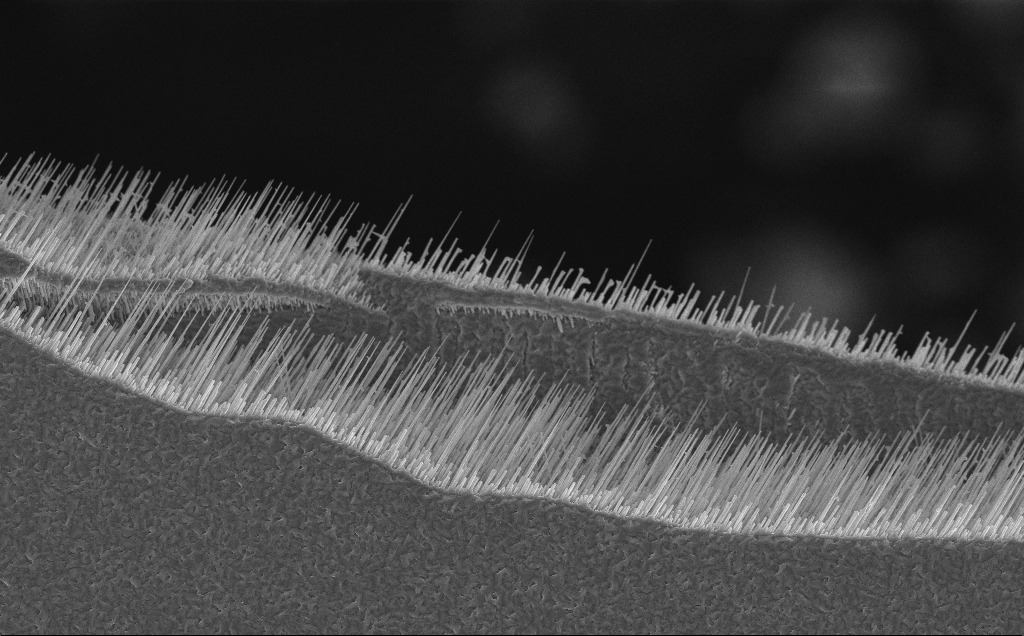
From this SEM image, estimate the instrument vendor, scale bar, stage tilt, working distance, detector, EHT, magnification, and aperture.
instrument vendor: Zeiss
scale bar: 10000 nm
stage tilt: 0°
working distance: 7 mm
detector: InLens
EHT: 10 kV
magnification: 5.26 K X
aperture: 30 µm